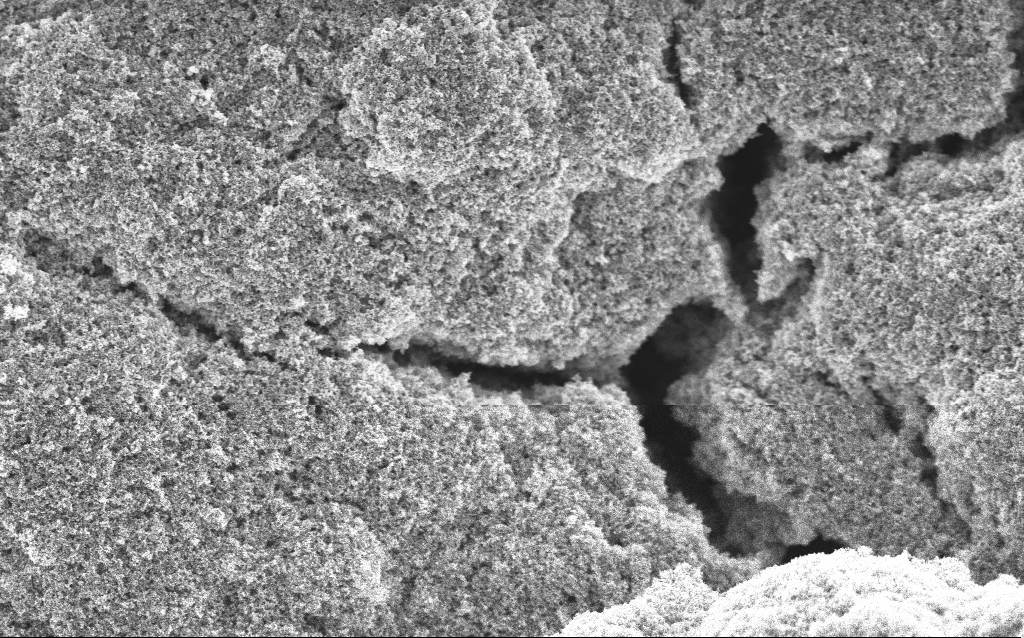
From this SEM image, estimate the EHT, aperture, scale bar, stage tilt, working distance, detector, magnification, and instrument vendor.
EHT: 5 kV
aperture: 30 µm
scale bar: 1000 nm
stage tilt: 0°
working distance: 4.2 mm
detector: InLens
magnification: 20.87 K X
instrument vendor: Zeiss